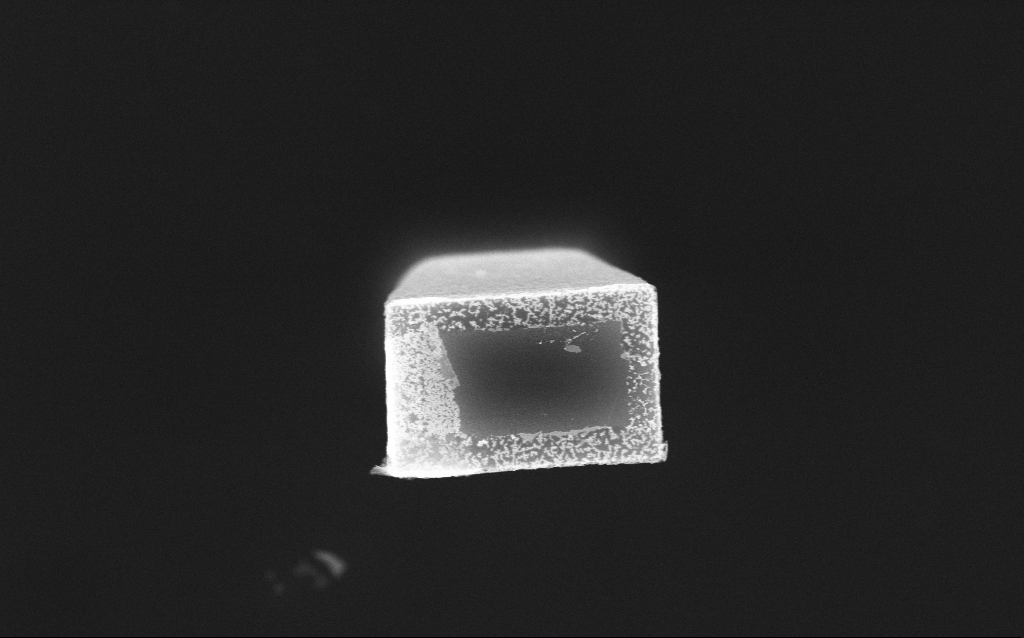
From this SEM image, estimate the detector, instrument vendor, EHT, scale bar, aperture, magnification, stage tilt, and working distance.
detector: InLens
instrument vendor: Zeiss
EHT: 10 kV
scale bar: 1000 nm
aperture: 30 µm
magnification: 34.52 K X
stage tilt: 0°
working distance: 8.4 mm